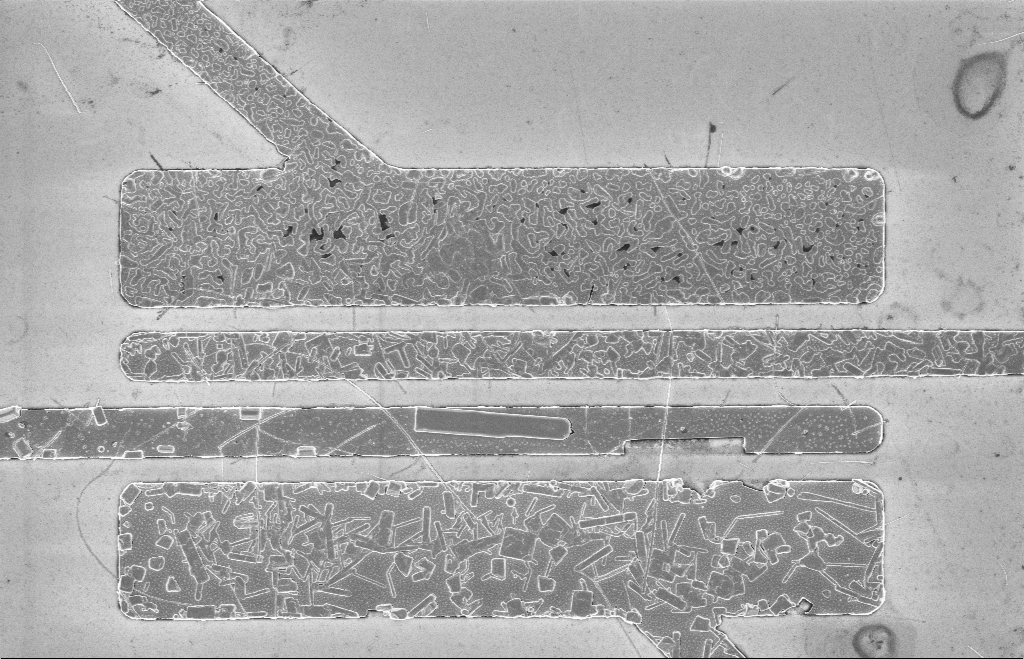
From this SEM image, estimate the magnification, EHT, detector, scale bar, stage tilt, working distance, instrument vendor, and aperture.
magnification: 4.64 K X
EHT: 5 kV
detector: InLens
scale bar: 10000 nm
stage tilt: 0°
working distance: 8 mm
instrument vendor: Zeiss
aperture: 20 µm